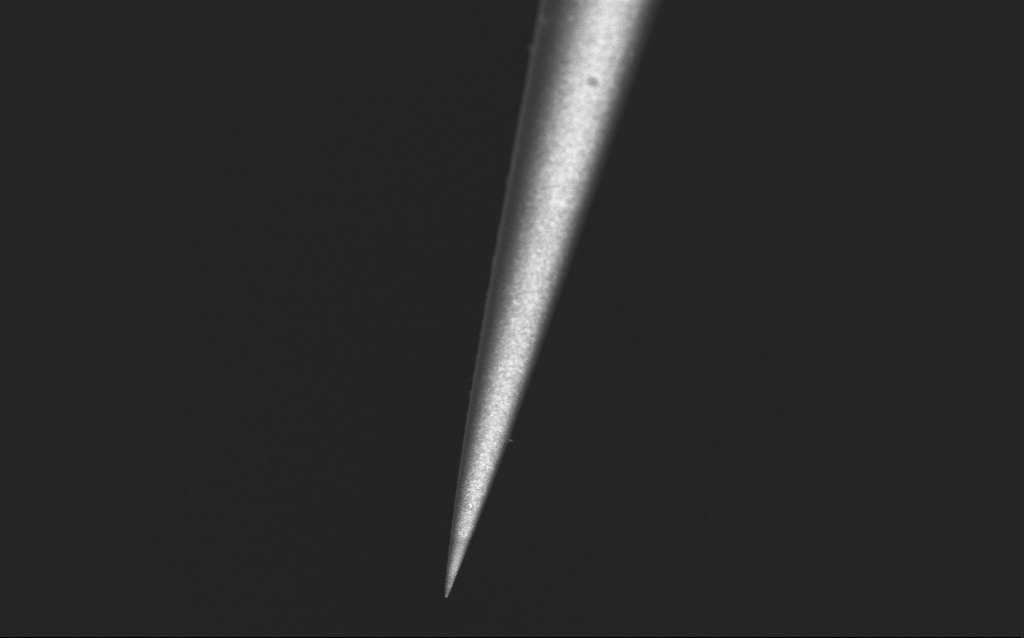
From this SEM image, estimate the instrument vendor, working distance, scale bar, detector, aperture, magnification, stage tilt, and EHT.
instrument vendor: Zeiss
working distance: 5 mm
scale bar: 2000 nm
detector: InLens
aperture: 30 µm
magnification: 10 K X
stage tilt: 45°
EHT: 2.5 kV